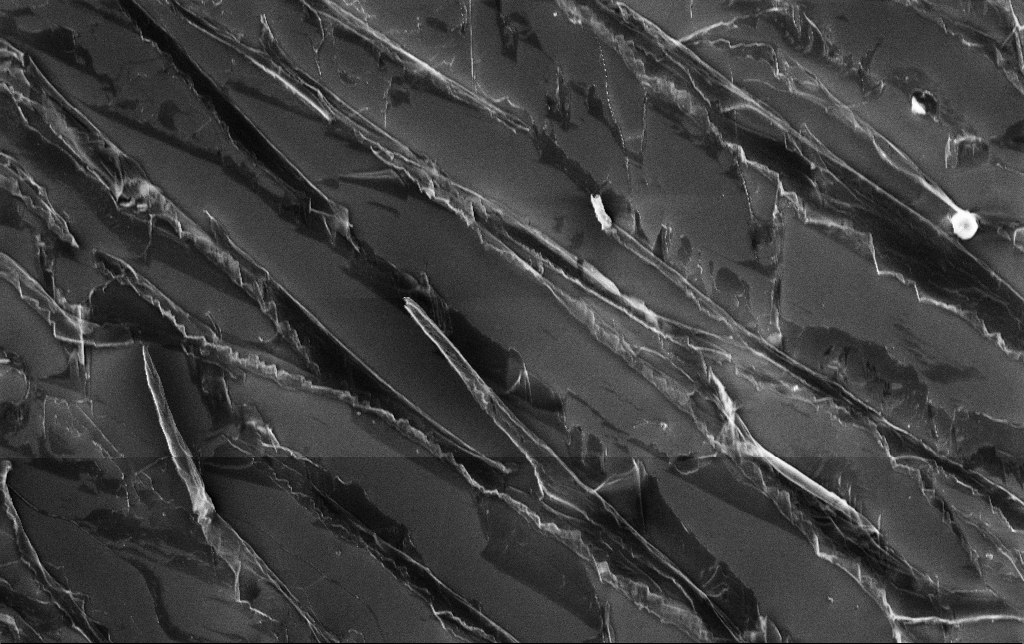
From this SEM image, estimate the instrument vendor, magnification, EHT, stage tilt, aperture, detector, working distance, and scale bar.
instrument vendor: Zeiss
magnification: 2.22 K X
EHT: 10 kV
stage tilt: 0°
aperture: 30 µm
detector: InLens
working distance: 3.2 mm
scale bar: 20000 nm